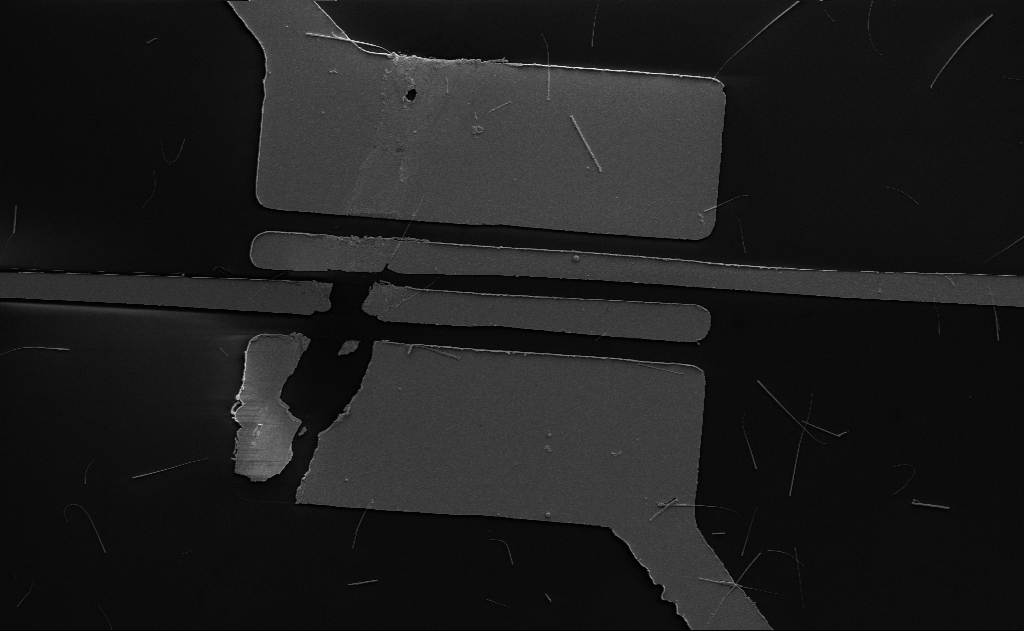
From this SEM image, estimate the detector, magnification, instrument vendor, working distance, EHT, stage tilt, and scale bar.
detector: SE2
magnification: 5.57 K X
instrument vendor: Zeiss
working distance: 15 mm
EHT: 5 kV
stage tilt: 0°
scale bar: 10000 nm